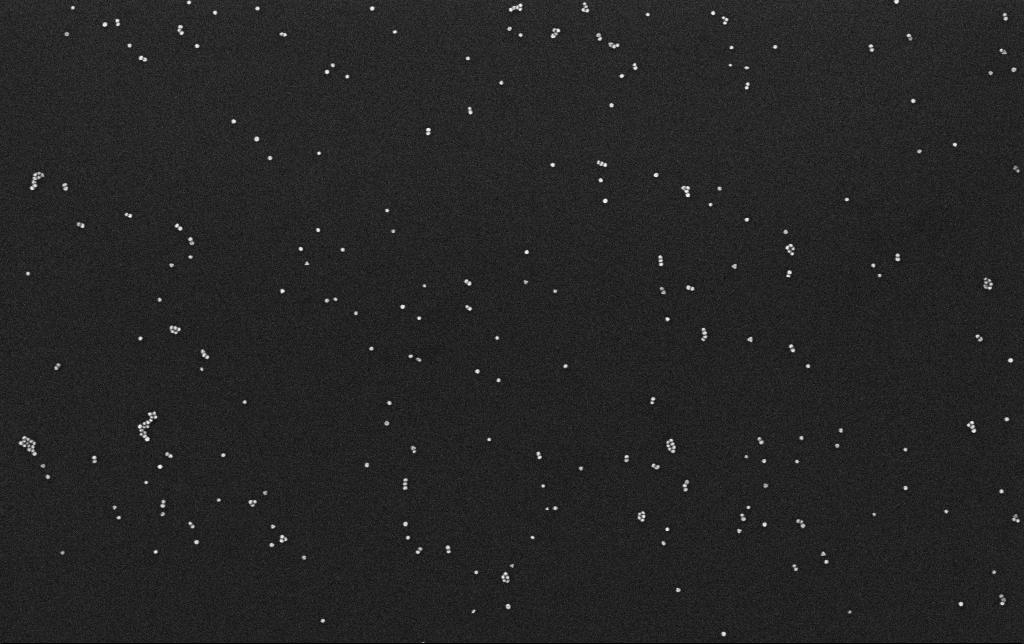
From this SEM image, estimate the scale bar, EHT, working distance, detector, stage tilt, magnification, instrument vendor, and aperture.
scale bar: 200 nm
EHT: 10 kV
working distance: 3.1 mm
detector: InLens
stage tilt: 0°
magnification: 100 K X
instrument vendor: Zeiss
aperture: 30 µm